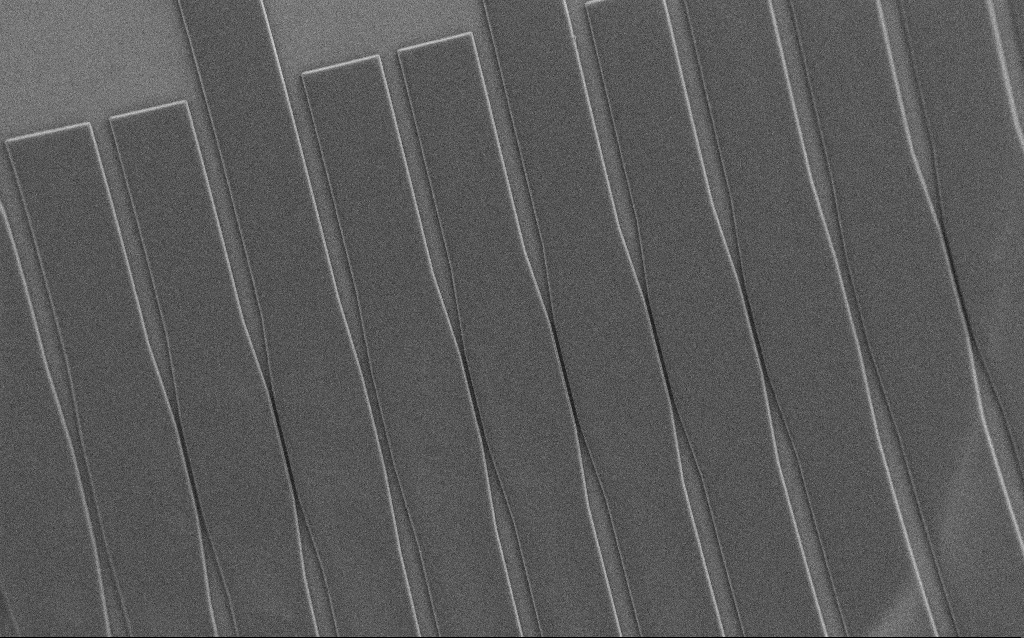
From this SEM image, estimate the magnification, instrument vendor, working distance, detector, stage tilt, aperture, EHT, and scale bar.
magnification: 0.332 K X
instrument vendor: Zeiss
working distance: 6 mm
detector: SE2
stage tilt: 0°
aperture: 30 µm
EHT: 1 kV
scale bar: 100000 nm